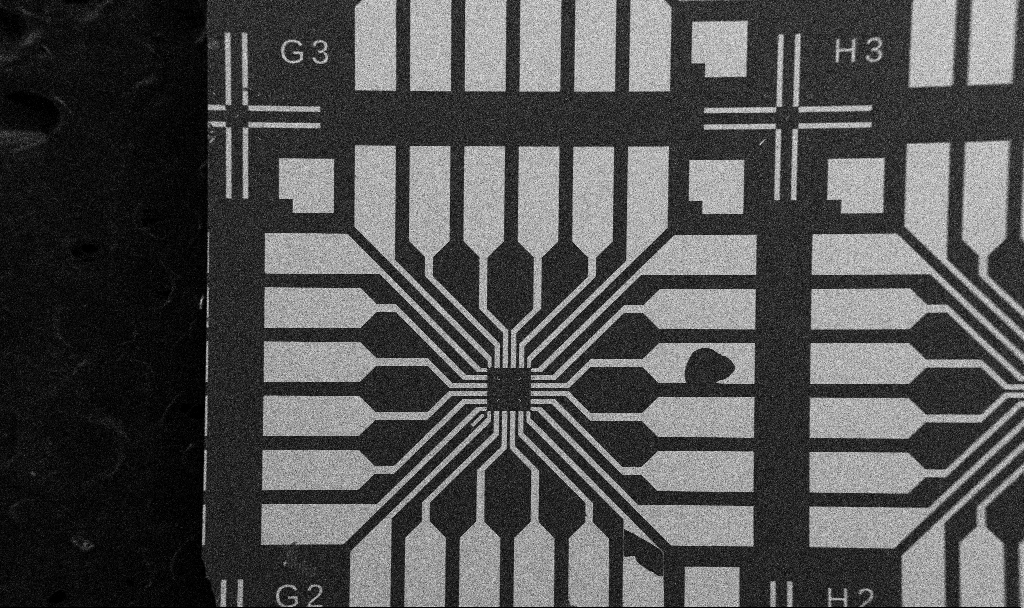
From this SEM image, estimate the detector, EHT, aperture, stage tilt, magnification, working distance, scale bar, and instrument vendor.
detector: SE2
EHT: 5 kV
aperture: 30 µm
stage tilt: -0°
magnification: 0.1 K X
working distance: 10.7 mm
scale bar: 200000 nm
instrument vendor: Zeiss